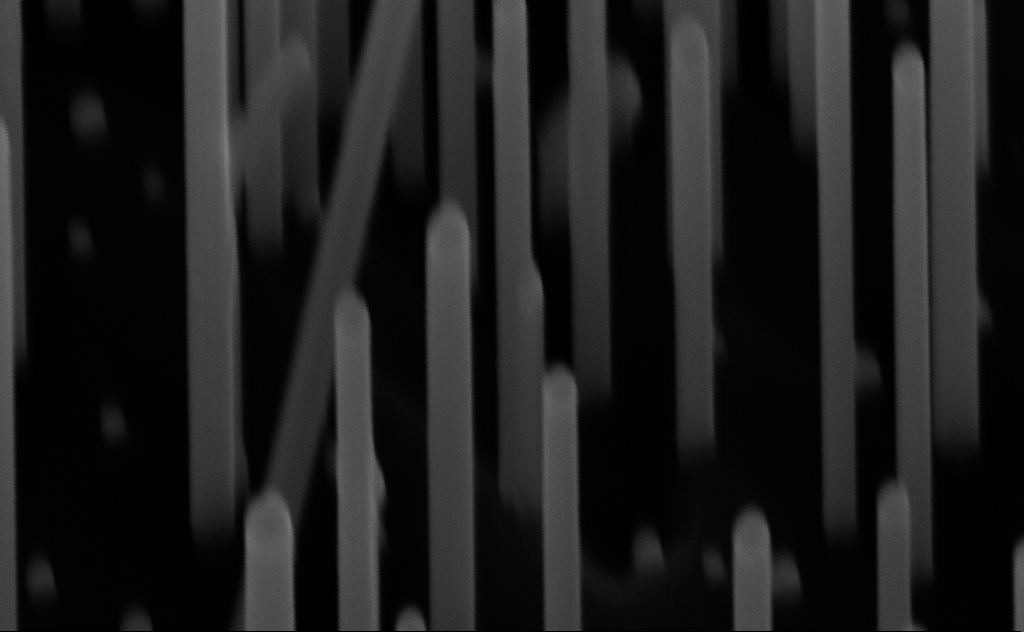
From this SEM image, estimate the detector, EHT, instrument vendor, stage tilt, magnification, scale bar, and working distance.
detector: InLens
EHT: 10 kV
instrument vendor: Zeiss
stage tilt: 45°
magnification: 241.67 K X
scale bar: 200 nm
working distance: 7 mm